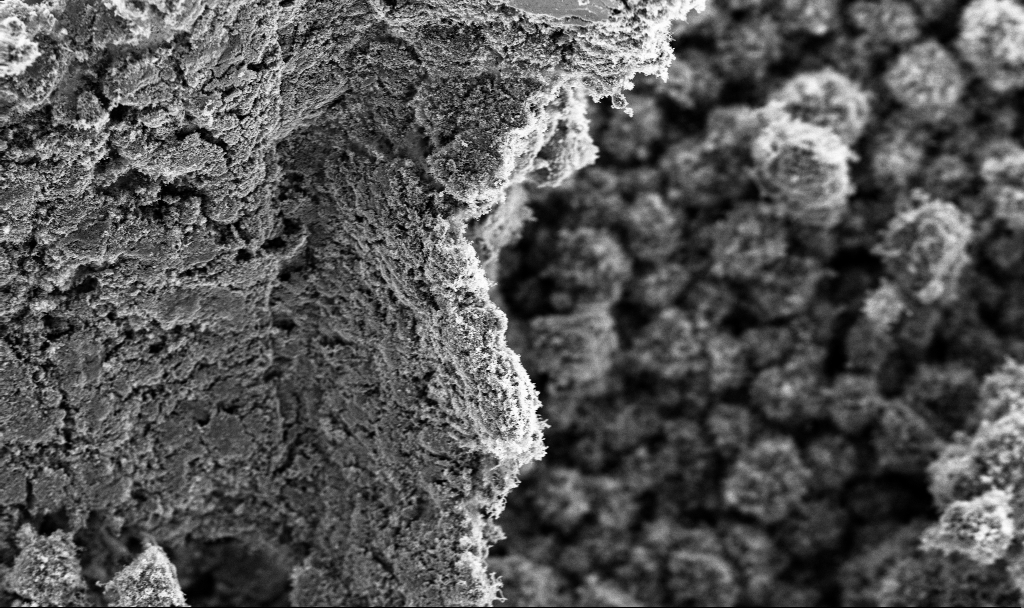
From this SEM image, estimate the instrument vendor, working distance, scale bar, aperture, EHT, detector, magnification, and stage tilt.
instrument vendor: Zeiss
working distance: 3.8 mm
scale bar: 10000 nm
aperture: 30 µm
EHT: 3 kV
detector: InLens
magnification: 5 K X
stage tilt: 0°